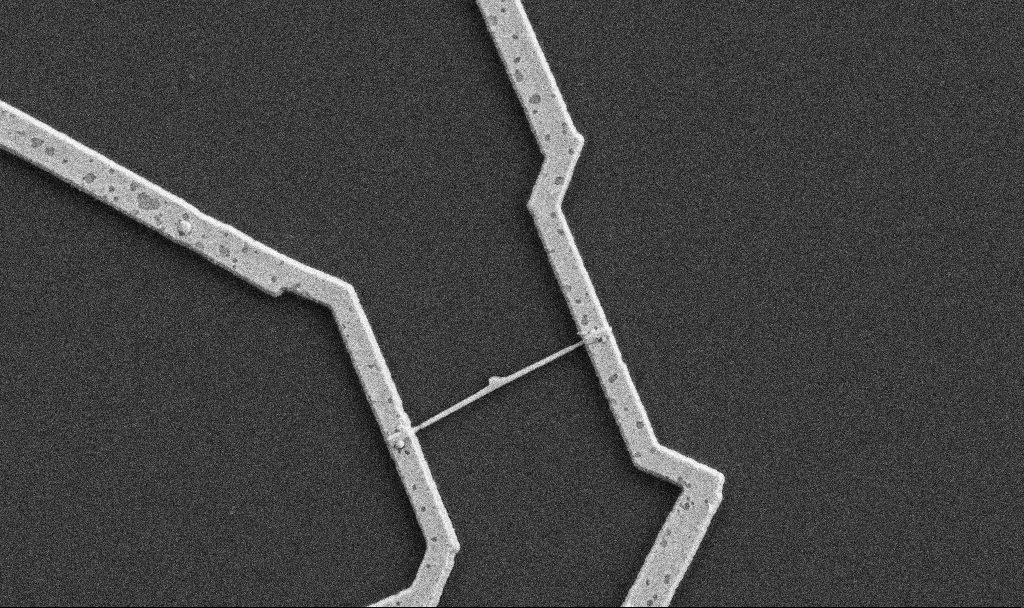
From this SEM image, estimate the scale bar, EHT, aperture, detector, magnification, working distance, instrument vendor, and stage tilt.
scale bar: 1000 nm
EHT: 5 kV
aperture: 30 µm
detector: SE2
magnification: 20 K X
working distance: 10.7 mm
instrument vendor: Zeiss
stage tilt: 0°